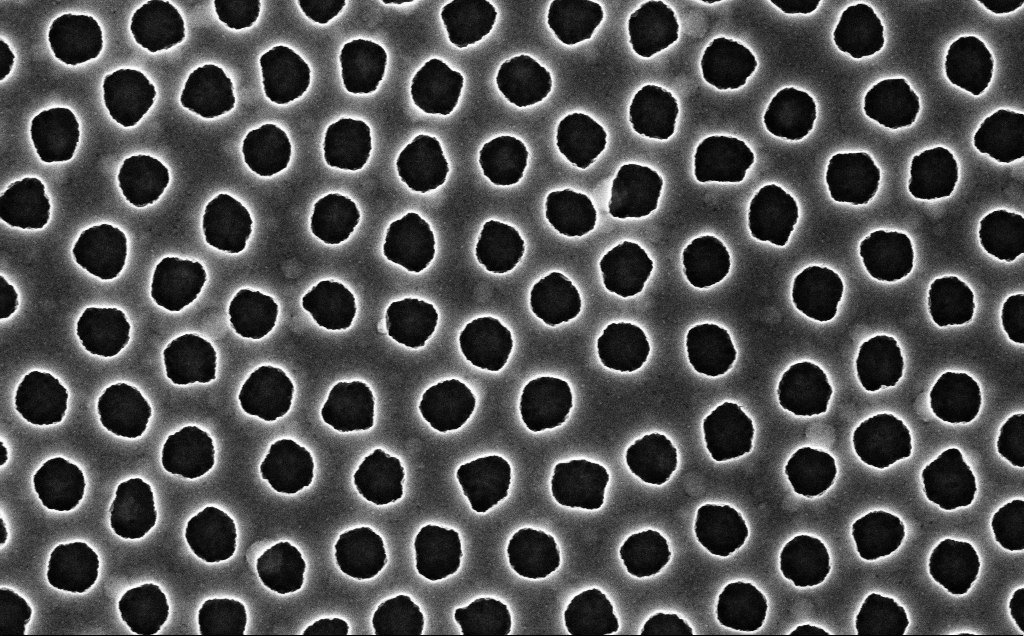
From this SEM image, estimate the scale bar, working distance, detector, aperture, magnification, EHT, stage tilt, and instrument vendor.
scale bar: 200 nm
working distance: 2.5 mm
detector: InLens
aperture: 30 µm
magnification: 90 K X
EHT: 3 kV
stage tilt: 0°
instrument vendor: Zeiss